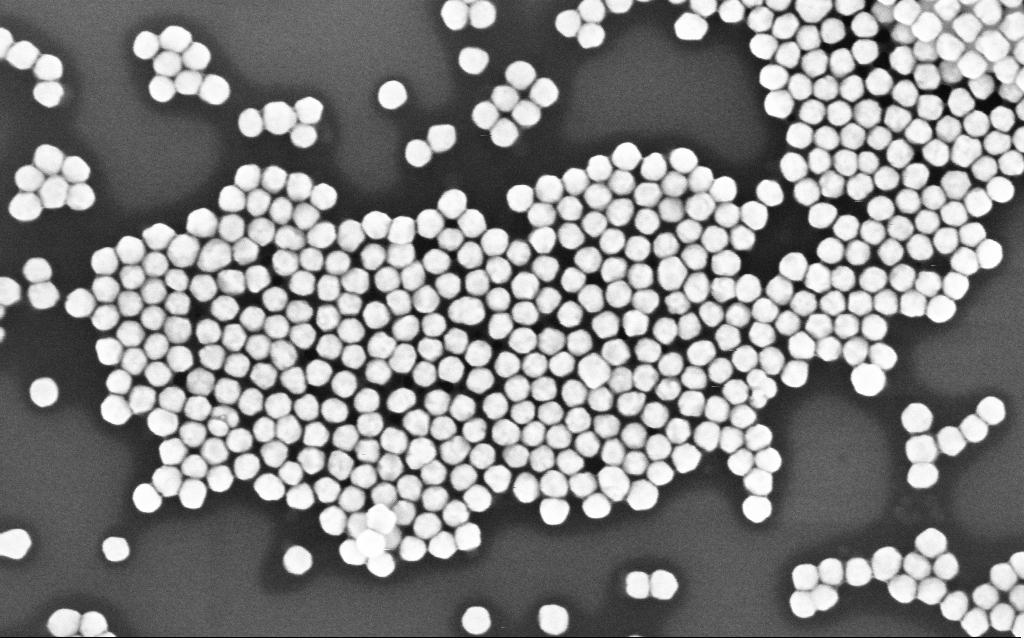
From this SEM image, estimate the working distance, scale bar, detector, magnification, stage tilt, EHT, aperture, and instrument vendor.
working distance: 3.1 mm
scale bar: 200 nm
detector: InLens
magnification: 164.55 K X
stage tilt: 0°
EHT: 8 kV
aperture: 30 µm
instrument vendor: Zeiss